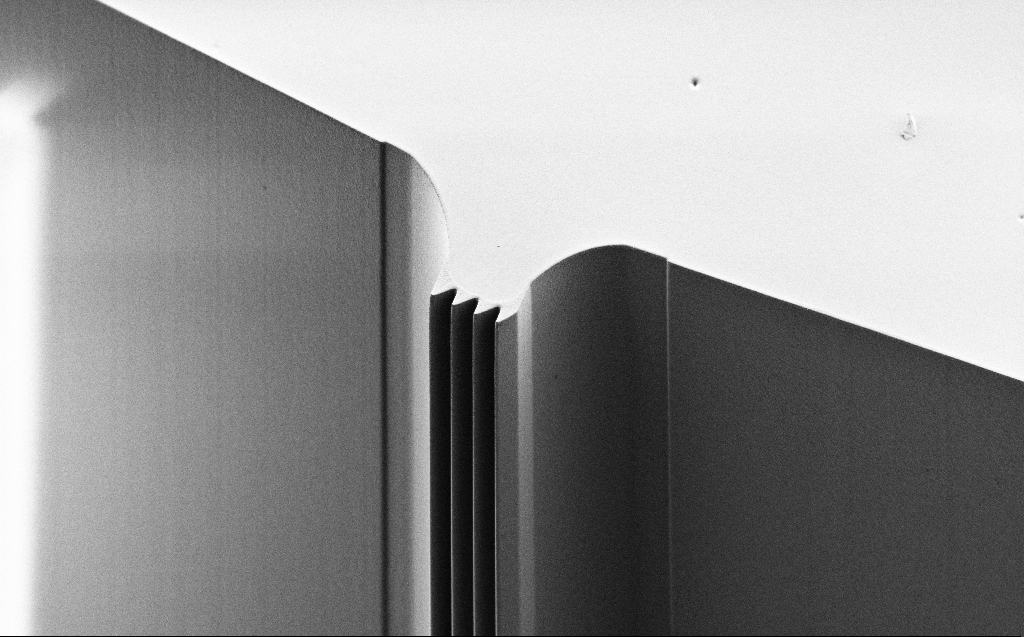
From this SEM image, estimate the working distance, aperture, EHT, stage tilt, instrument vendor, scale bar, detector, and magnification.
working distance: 6 mm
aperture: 30 µm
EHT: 0.9 kV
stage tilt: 45°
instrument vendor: Zeiss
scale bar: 20000 nm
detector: SE2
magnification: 0.769 K X